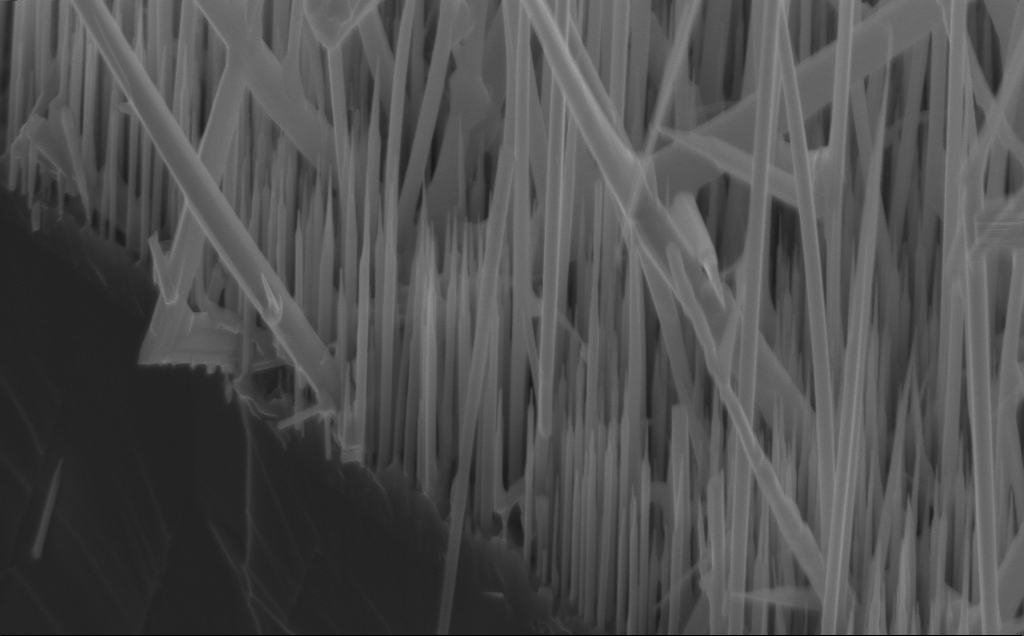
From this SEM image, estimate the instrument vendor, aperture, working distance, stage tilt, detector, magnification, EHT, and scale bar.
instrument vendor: Zeiss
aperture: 30 µm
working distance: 5 mm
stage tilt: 45°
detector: InLens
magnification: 44.96 K X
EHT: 10 kV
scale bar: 1000 nm